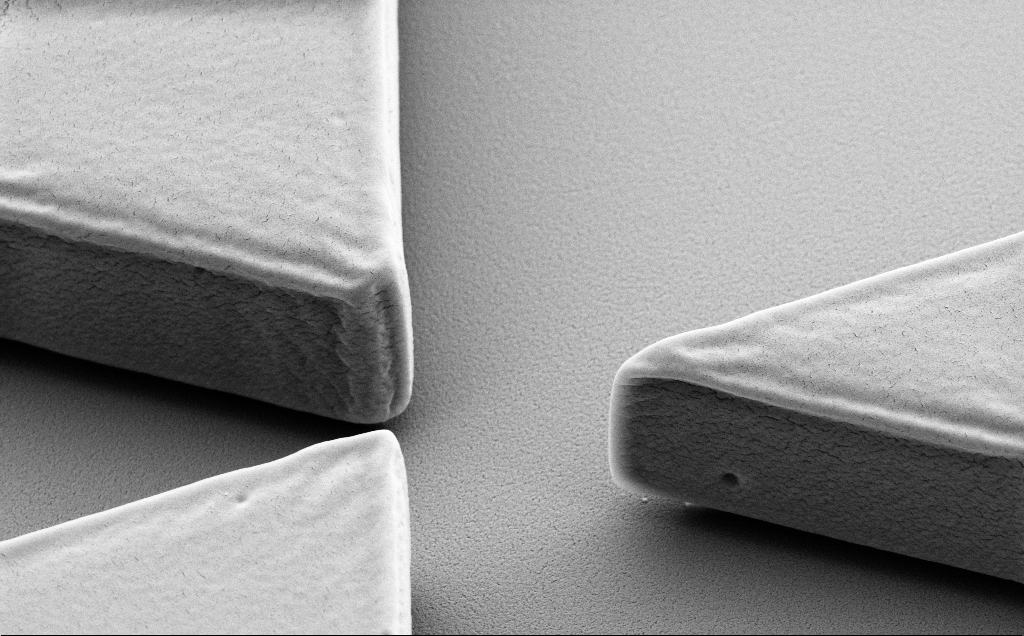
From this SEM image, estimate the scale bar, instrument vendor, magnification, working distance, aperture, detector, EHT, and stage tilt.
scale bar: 2000 nm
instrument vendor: Zeiss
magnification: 16.97 K X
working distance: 9 mm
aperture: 30 µm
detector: SE2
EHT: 5 kV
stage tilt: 40°